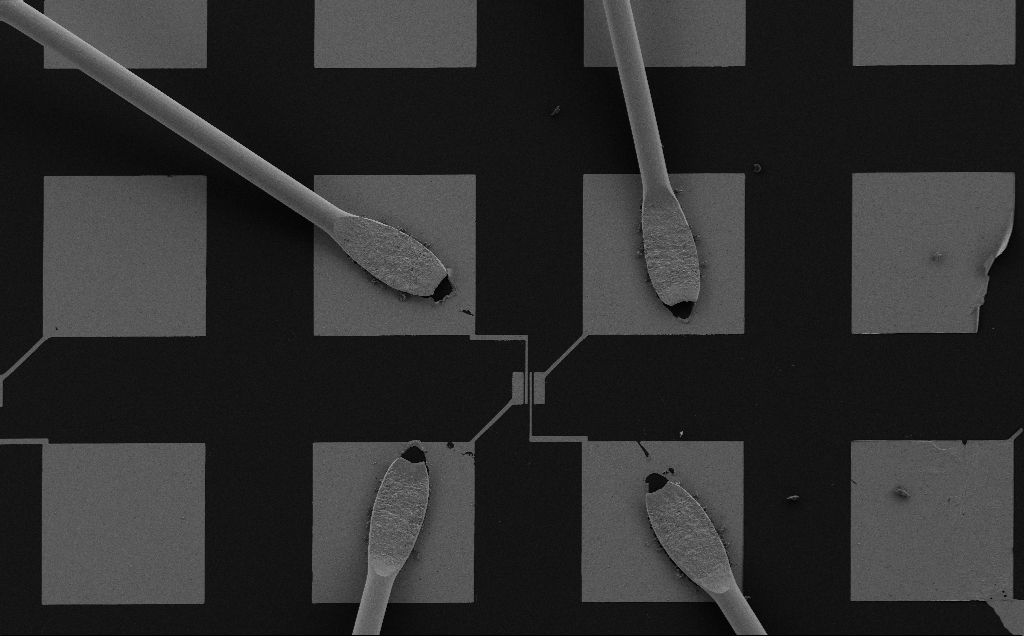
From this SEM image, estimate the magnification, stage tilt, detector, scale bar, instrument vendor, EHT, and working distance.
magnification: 0.398 K X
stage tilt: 0°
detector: SE2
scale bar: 100000 nm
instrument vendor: Zeiss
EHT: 5 kV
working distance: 6 mm